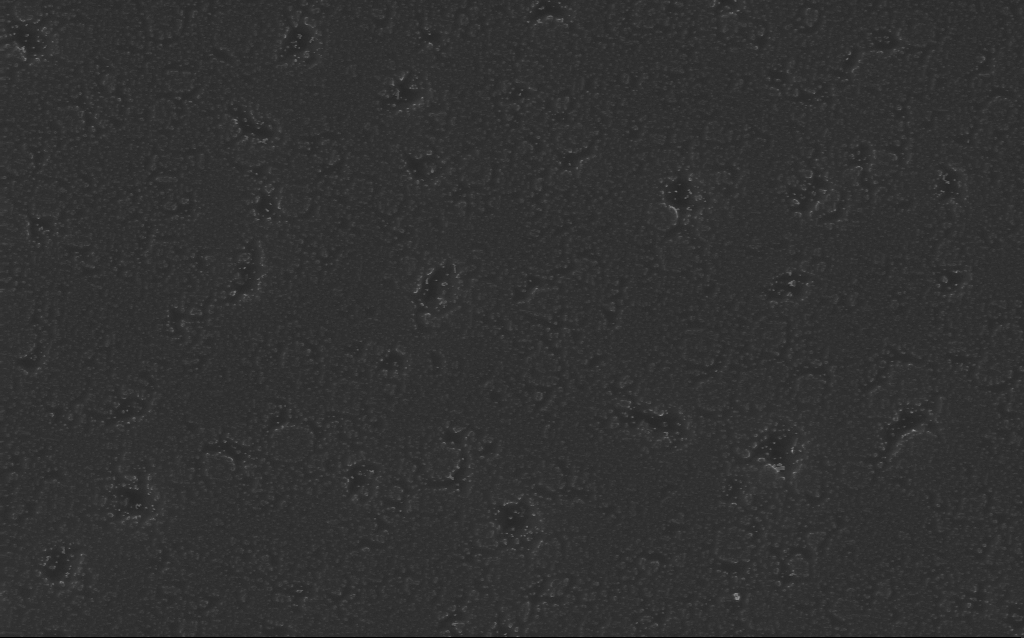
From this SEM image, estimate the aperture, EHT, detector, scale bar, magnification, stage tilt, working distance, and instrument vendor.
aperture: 30 µm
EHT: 10 kV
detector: InLens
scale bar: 2000 nm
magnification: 32.35 K X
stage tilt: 0°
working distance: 5 mm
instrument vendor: Zeiss